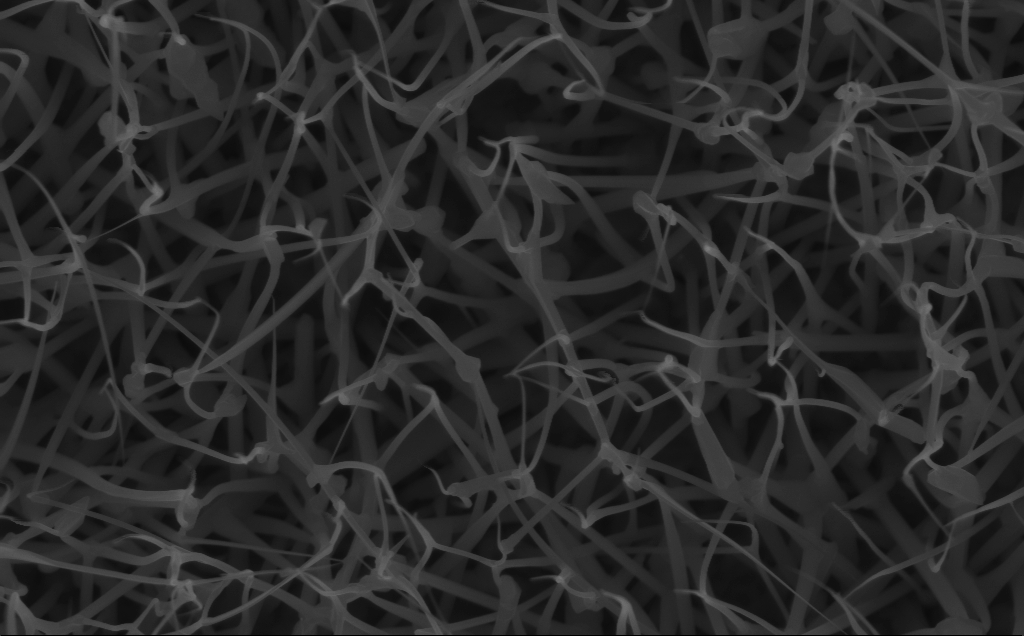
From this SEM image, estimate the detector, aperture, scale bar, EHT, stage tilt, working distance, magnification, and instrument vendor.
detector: InLens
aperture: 30 µm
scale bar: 1000 nm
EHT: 10 kV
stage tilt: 0°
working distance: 6 mm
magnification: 40 K X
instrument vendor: Zeiss